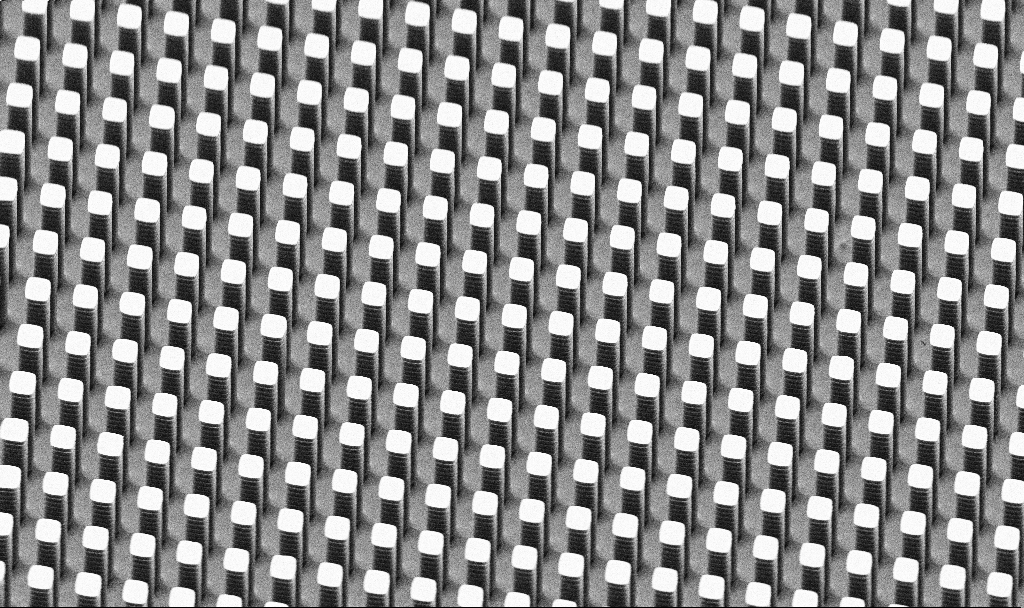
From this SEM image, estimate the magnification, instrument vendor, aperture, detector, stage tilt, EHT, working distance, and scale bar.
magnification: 4.37 K X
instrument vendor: Zeiss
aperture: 30 µm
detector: SE2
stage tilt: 45°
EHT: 5 kV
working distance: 7.5 mm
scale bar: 10000 nm